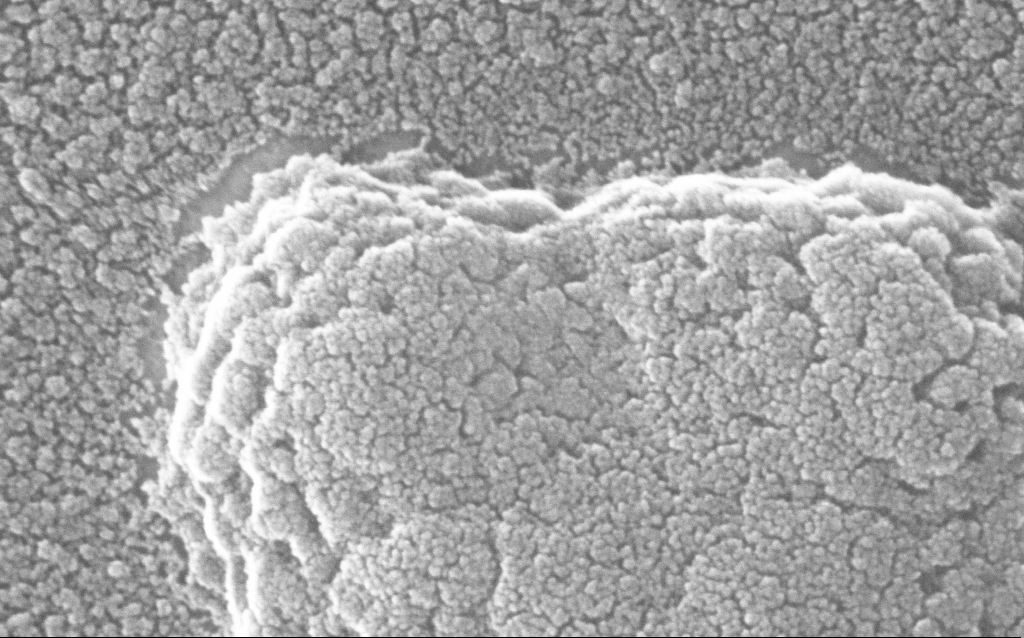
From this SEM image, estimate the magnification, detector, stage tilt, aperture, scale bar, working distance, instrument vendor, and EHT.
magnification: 500 K X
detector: InLens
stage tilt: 0°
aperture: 30 µm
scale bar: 100 nm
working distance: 1.8 mm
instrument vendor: Zeiss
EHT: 20 kV